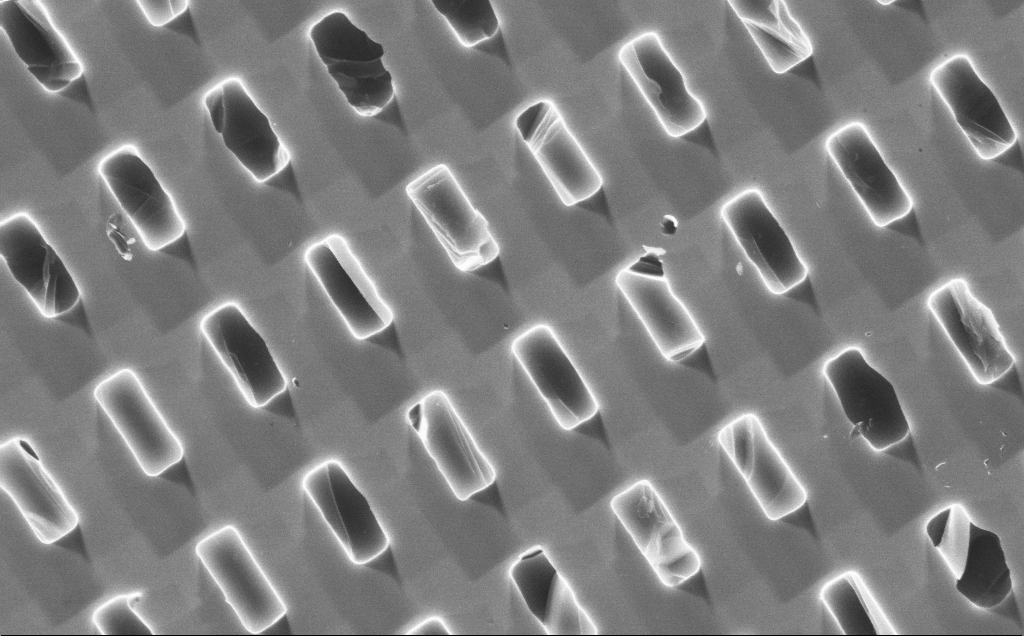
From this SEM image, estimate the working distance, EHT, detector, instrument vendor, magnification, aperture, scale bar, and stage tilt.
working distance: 9 mm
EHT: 10 kV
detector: InLens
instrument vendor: Zeiss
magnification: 11.58 K X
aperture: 30 µm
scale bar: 2000 nm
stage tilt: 0°